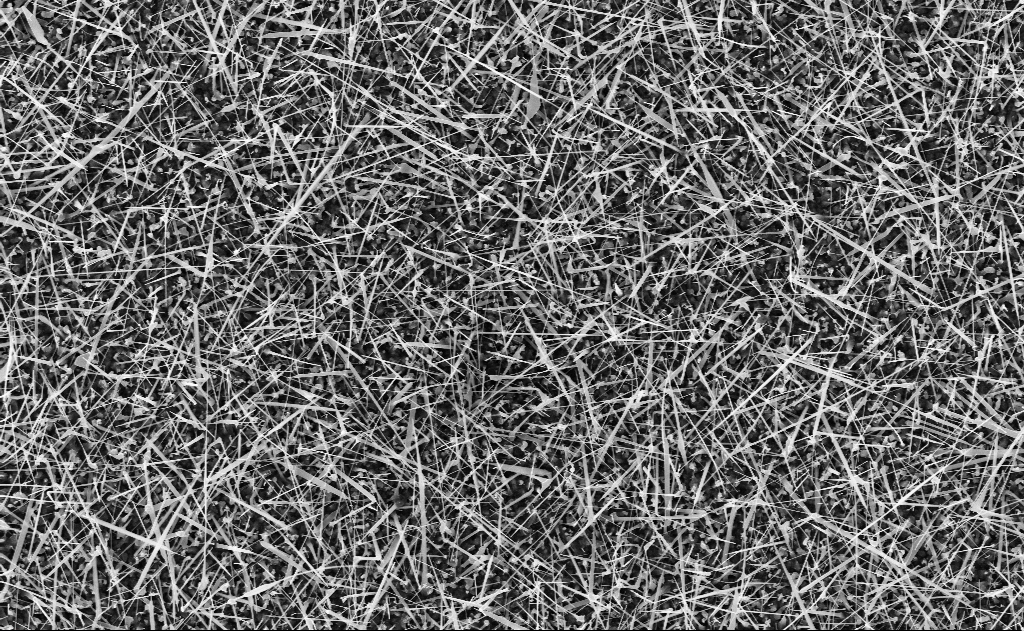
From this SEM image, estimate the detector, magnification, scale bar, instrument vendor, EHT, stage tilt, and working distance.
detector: InLens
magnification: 10 K X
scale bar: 2000 nm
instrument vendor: Zeiss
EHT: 10 kV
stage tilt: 0°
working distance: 11 mm